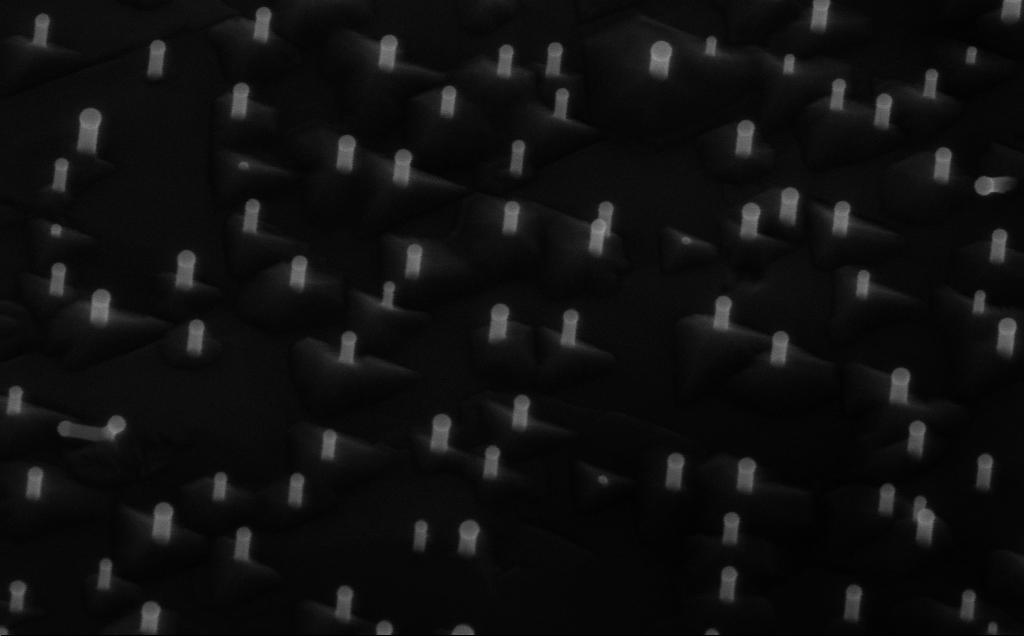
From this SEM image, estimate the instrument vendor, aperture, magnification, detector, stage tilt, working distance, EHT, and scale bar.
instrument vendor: Zeiss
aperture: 30 µm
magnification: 150 K X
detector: InLens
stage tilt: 45°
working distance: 6 mm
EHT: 10 kV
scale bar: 100 nm